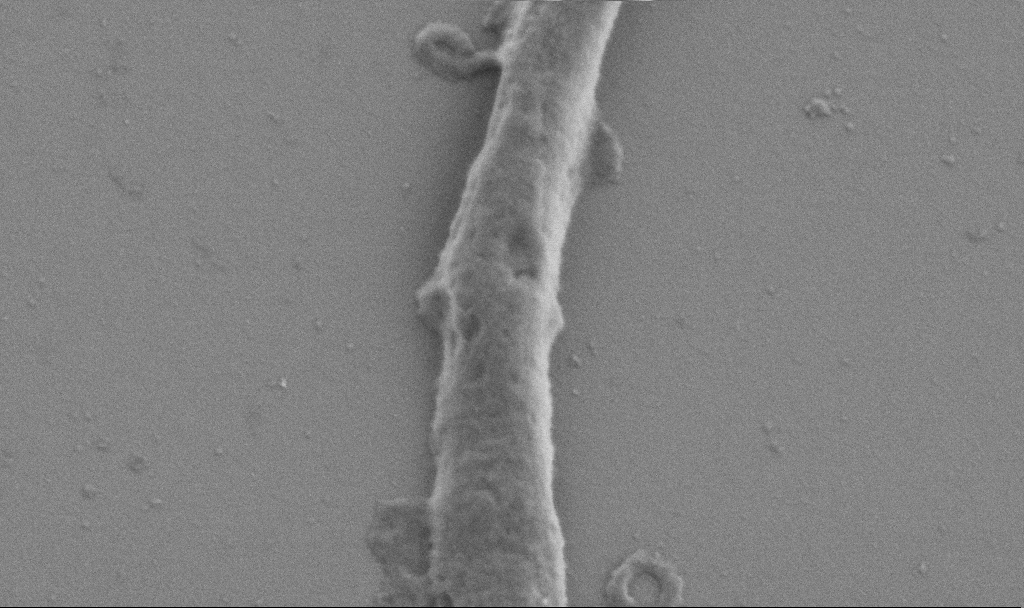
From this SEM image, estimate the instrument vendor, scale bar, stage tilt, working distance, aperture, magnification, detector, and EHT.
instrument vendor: Zeiss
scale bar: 1000 nm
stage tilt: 0°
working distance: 6.9 mm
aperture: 30 µm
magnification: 50 K X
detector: SE2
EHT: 0.9 kV